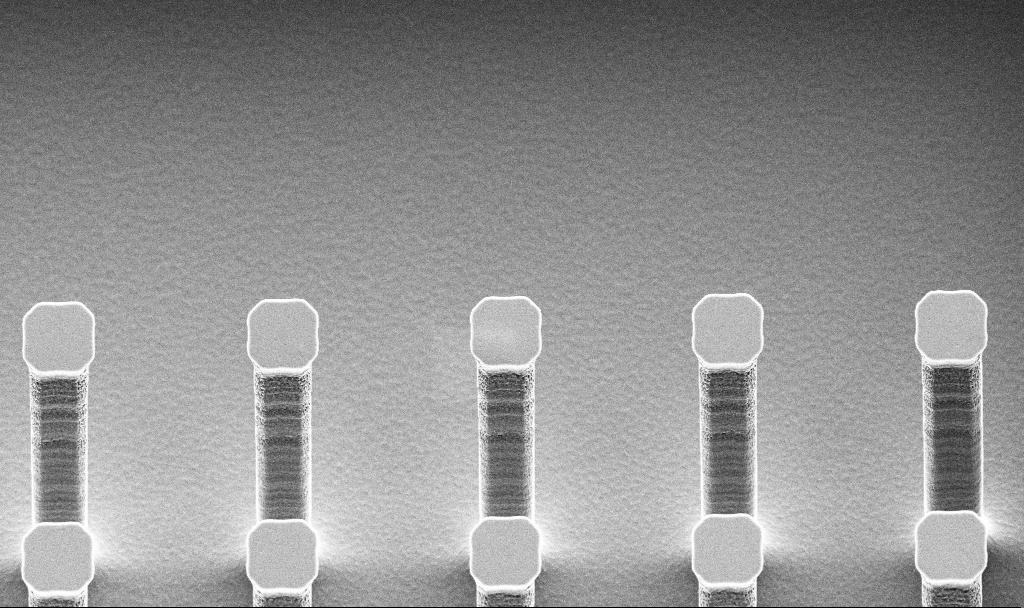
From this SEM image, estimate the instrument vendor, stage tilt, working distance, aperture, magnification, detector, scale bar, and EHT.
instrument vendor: Zeiss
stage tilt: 45°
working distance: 13.7 mm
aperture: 30 µm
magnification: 2.72 K X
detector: SE2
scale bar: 20000 nm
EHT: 5 kV